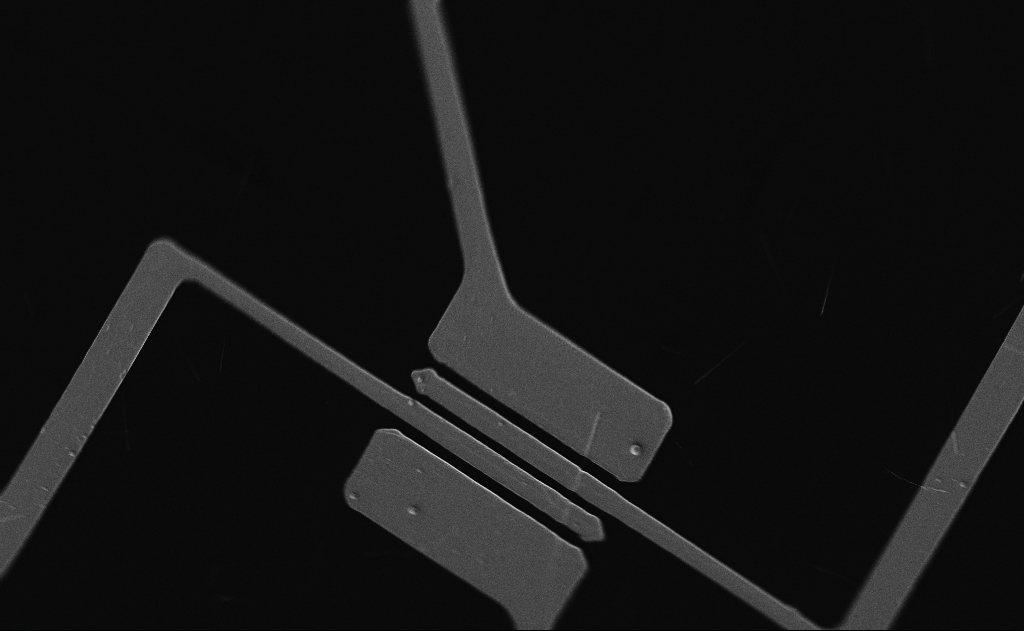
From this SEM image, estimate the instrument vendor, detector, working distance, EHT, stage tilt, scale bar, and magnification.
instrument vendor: Zeiss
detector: InLens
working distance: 21 mm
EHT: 5 kV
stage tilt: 0°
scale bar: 20000 nm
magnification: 3 K X